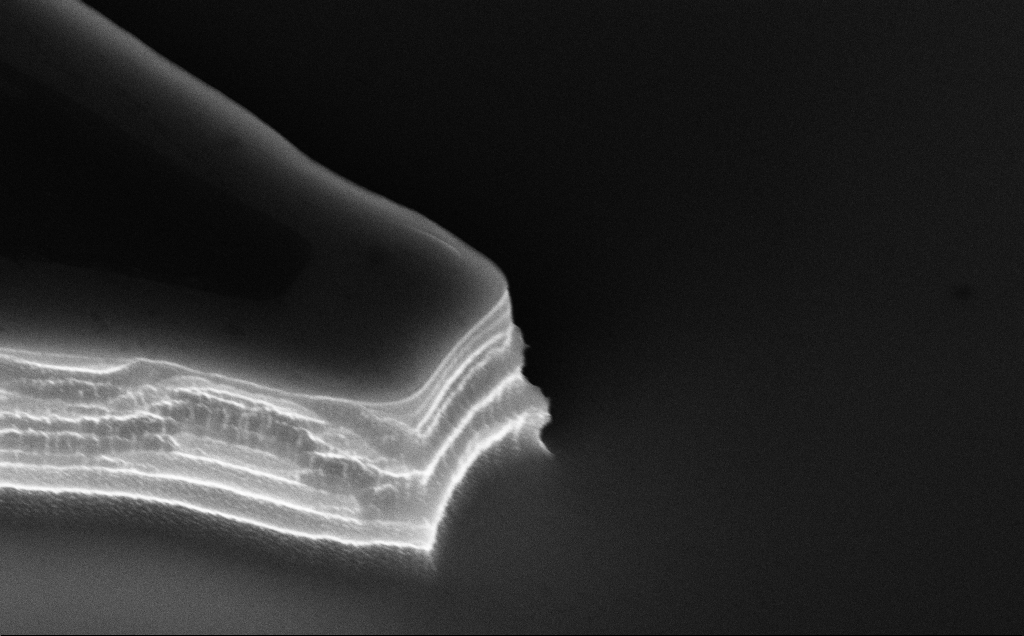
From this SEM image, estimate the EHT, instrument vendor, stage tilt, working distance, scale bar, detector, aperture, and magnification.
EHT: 10 kV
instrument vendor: Zeiss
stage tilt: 50°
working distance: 10 mm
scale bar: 1000 nm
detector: InLens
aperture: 30 µm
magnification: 31.8 K X